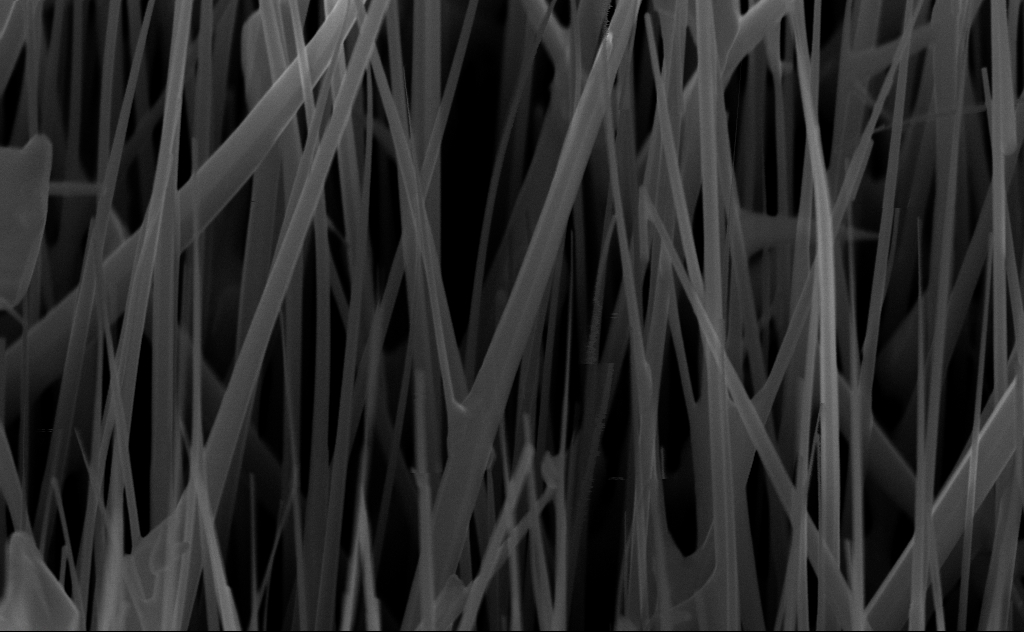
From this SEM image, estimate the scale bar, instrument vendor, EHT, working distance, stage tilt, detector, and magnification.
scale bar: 200 nm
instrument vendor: Zeiss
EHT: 10 kV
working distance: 6 mm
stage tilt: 45°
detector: InLens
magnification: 80 K X